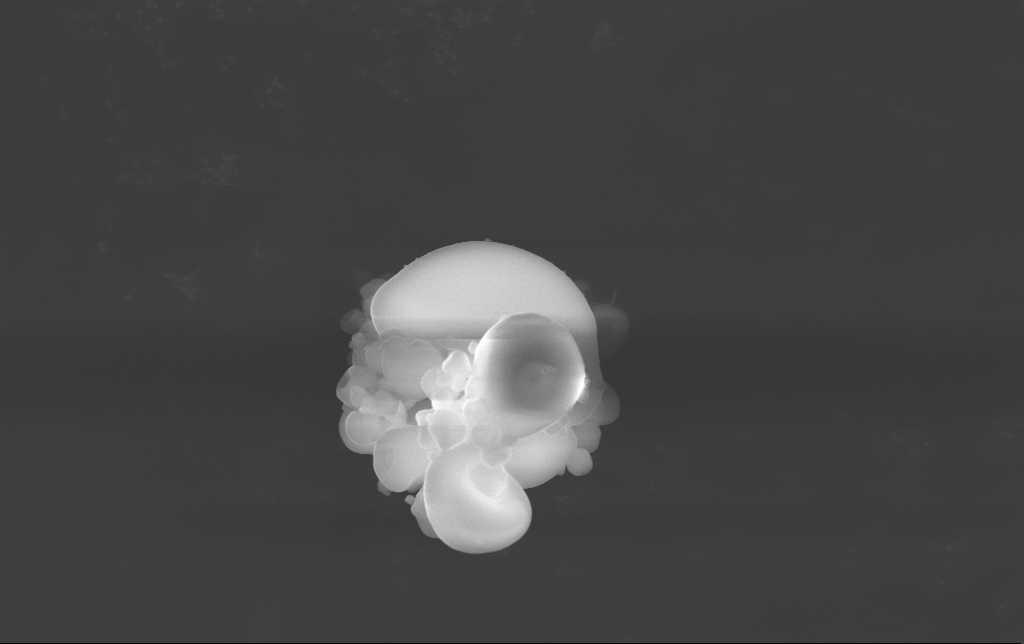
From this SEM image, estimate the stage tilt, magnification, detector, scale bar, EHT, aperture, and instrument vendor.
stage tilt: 0°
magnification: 10.41 K X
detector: InLens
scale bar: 2000 nm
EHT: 15 kV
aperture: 30 µm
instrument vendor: Zeiss